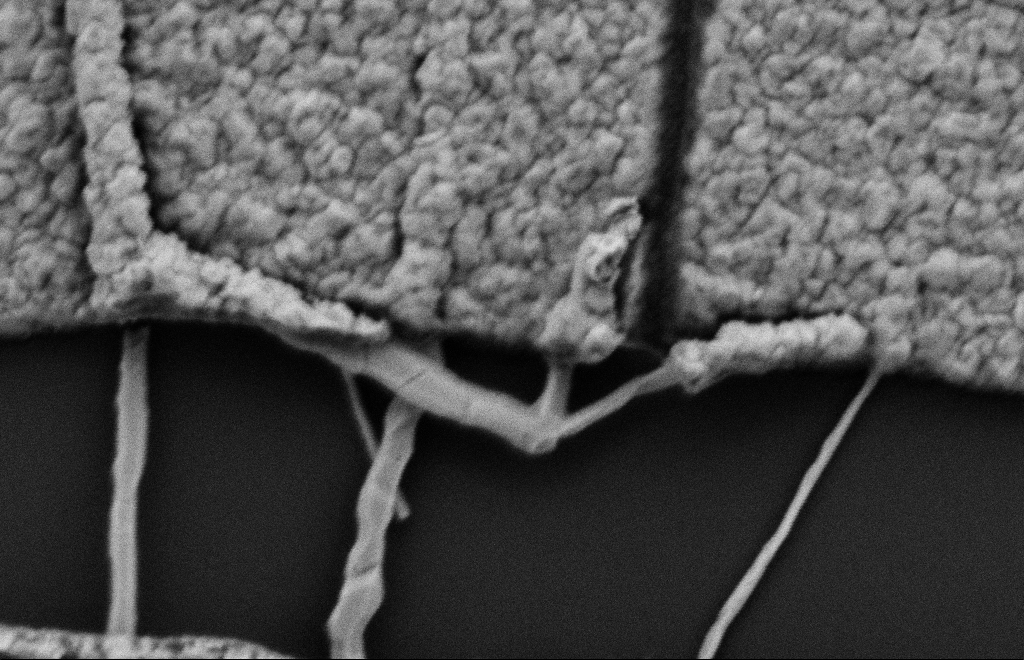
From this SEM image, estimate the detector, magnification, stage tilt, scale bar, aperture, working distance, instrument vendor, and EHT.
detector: SE2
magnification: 86.35 K X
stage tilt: -0.3°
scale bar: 200 nm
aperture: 20 µm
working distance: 10 mm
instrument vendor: Zeiss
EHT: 2 kV